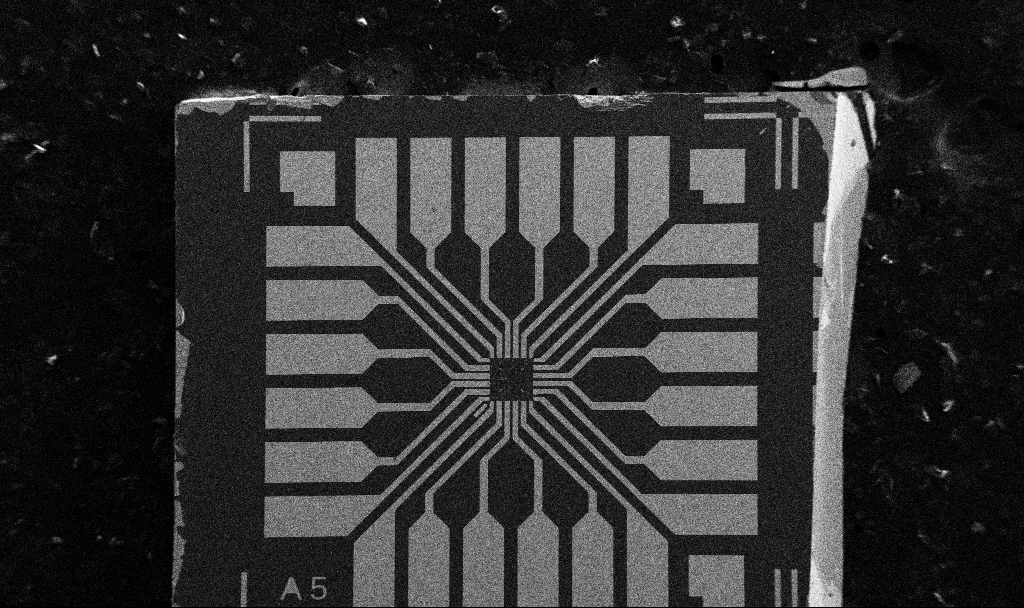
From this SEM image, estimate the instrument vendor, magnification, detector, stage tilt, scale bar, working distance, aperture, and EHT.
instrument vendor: Zeiss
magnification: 0.1 K X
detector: SE2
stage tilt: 0°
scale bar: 200000 nm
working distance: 10.7 mm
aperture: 30 µm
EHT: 5 kV